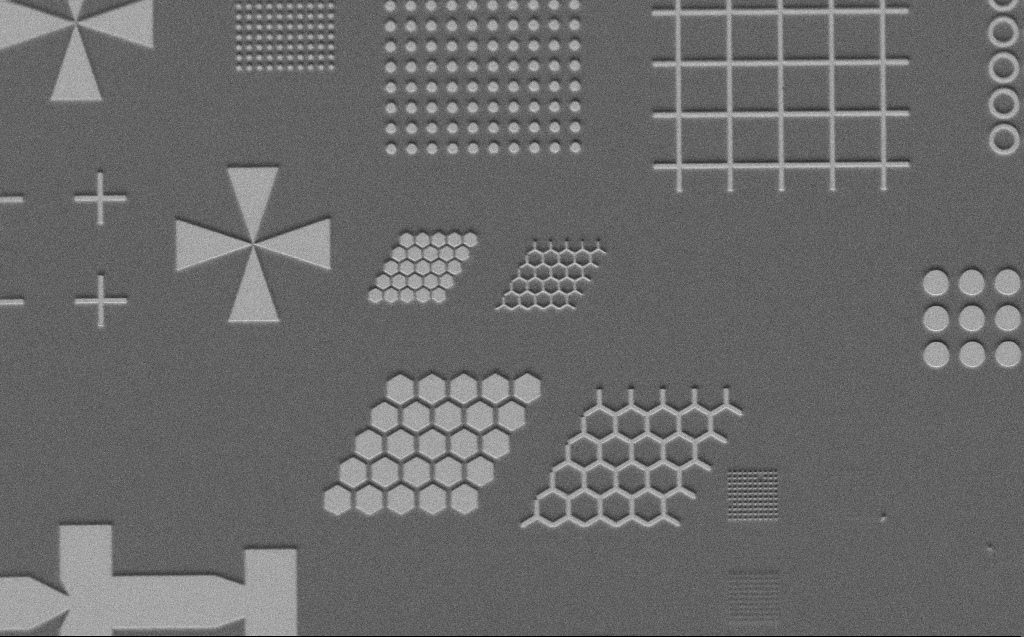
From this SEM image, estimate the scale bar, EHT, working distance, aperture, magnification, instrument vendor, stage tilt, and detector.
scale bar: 10000 nm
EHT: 3 kV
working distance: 6 mm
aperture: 30 µm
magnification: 1.89 K X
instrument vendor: Zeiss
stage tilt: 45°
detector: SE2